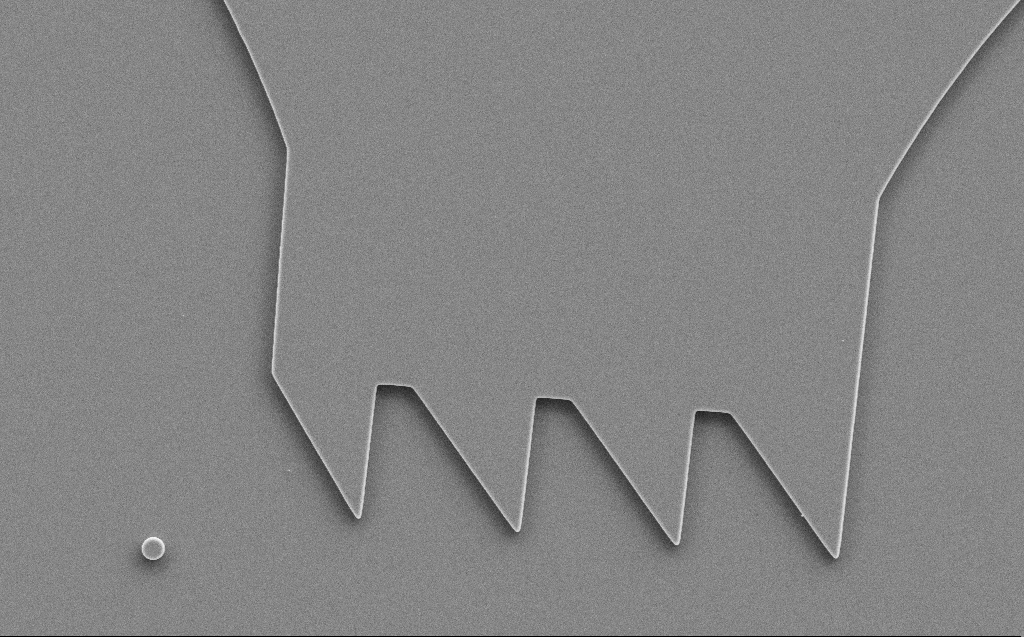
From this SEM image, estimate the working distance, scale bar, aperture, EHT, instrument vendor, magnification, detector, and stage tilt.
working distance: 6 mm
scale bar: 10000 nm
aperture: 30 µm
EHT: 5 kV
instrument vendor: Zeiss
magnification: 5.19 K X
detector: SE2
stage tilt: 0°